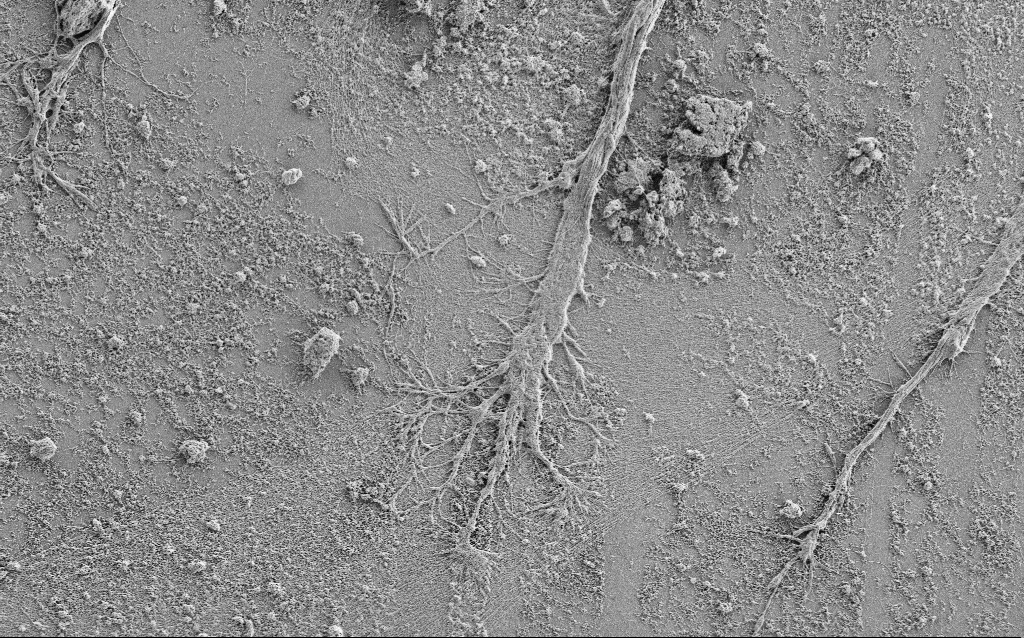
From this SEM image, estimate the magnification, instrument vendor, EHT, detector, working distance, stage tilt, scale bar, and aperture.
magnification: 2.5 K X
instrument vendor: Zeiss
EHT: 1 kV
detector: SE2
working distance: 4 mm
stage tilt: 0°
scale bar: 10000 nm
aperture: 30 µm